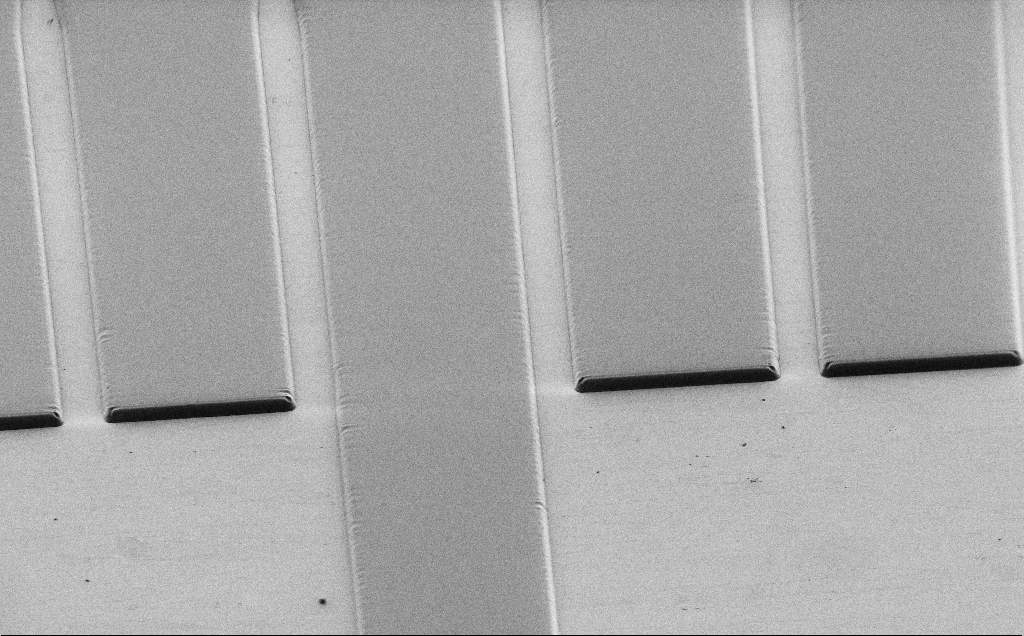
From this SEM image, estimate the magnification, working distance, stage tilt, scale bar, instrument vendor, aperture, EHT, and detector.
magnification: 0.823 K X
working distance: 8 mm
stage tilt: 45°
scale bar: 20000 nm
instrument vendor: Zeiss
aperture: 30 µm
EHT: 1 kV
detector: SE2